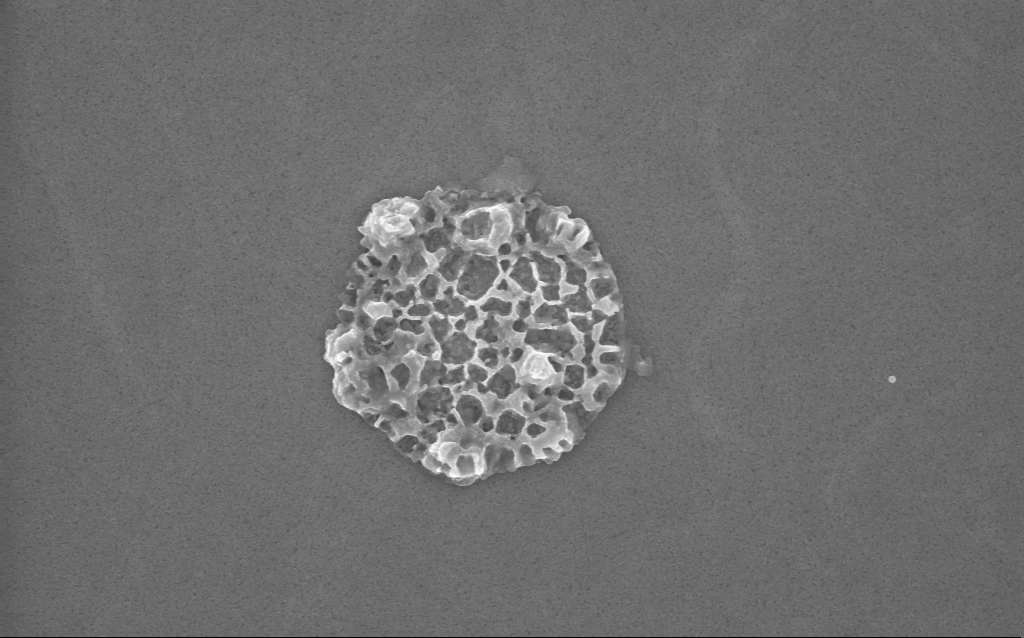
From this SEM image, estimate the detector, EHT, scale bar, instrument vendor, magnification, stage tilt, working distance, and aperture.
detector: InLens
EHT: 10 kV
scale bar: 1000 nm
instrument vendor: Zeiss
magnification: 68.79 K X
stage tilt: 0°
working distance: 2 mm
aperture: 30 µm